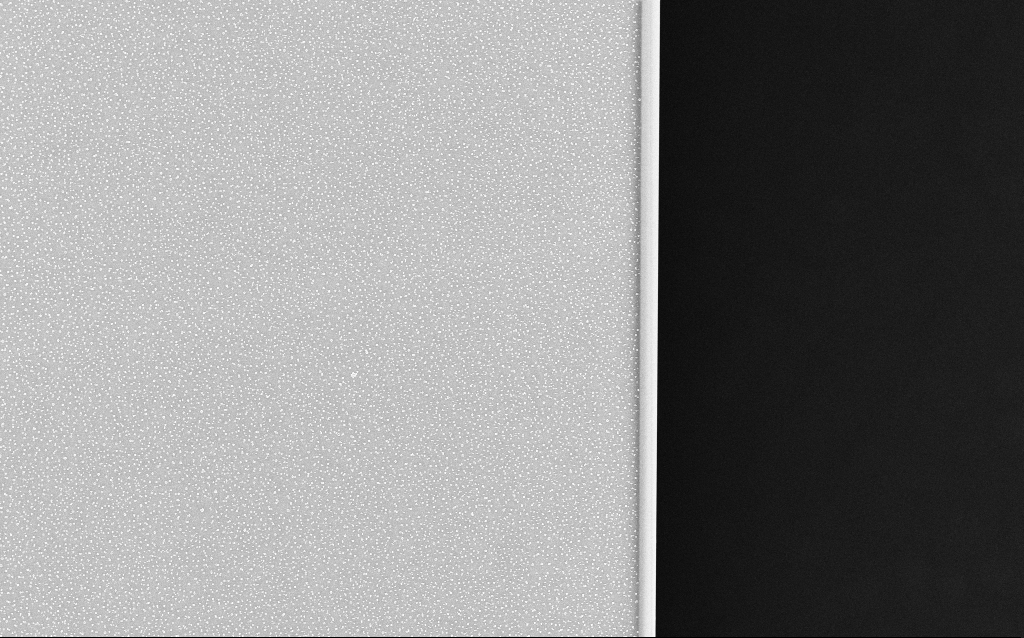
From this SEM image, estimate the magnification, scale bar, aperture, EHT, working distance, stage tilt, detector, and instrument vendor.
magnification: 1 K X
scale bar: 20000 nm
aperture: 30 µm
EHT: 20 kV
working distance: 3.4 mm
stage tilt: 0°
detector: InLens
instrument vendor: Zeiss